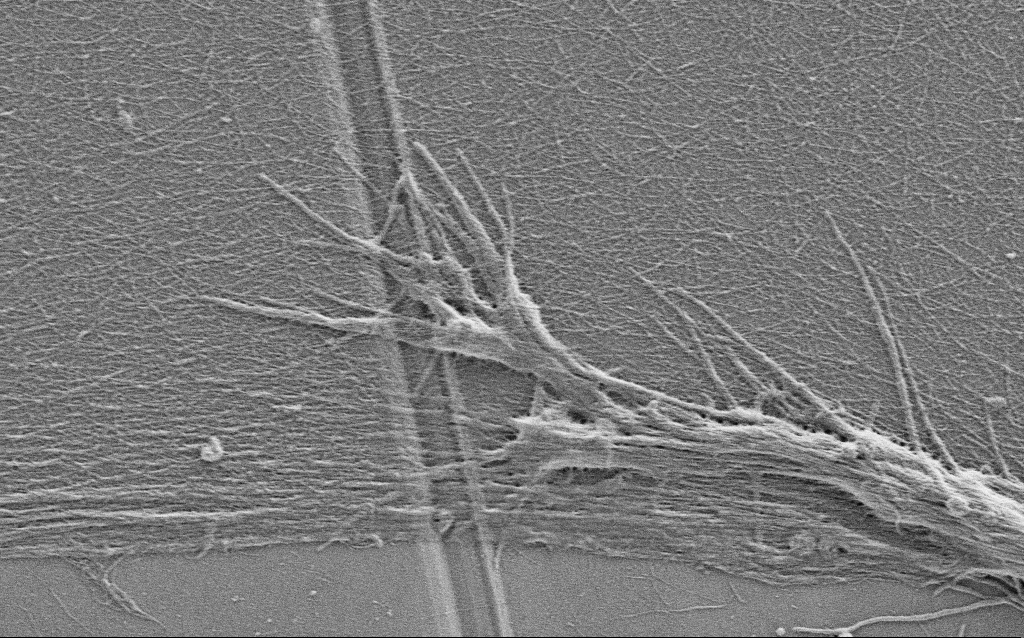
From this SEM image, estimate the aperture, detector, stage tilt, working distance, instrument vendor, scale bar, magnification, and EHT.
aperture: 30 µm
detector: SE2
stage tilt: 0°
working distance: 4 mm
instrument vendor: Zeiss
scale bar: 2000 nm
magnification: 15 K X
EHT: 0.9 kV